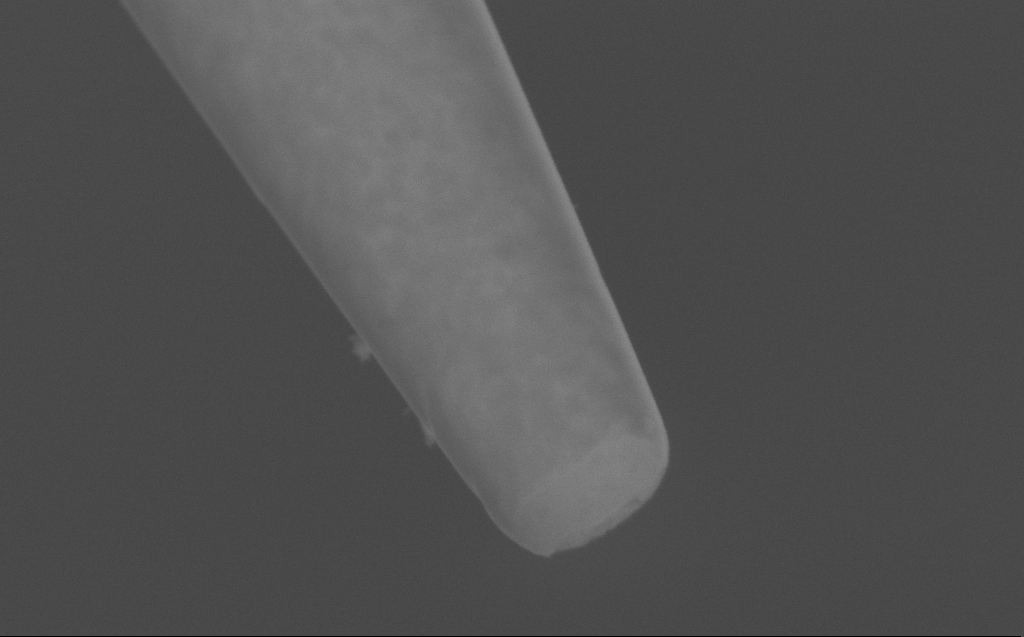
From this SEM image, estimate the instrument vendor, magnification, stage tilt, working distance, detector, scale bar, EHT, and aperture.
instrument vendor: Zeiss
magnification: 88.71 K X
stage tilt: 45°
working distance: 4 mm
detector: SE2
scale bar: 200 nm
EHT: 5 kV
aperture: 30 µm